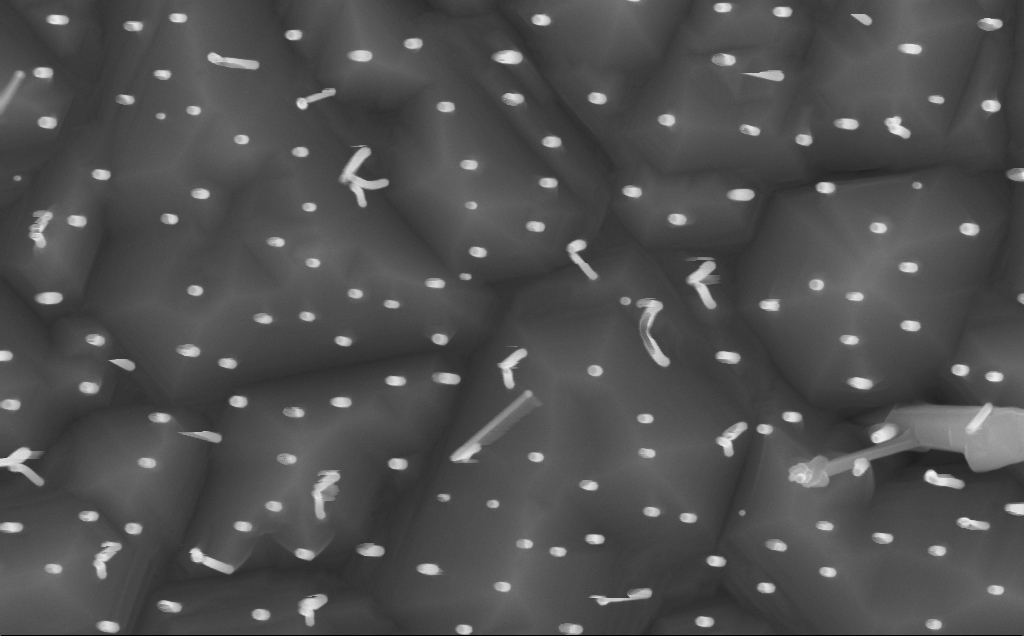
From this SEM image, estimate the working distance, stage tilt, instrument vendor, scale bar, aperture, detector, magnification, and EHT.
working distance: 7 mm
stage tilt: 0.2°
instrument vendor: Zeiss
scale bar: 200 nm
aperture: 30 µm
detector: InLens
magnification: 80 K X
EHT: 10 kV